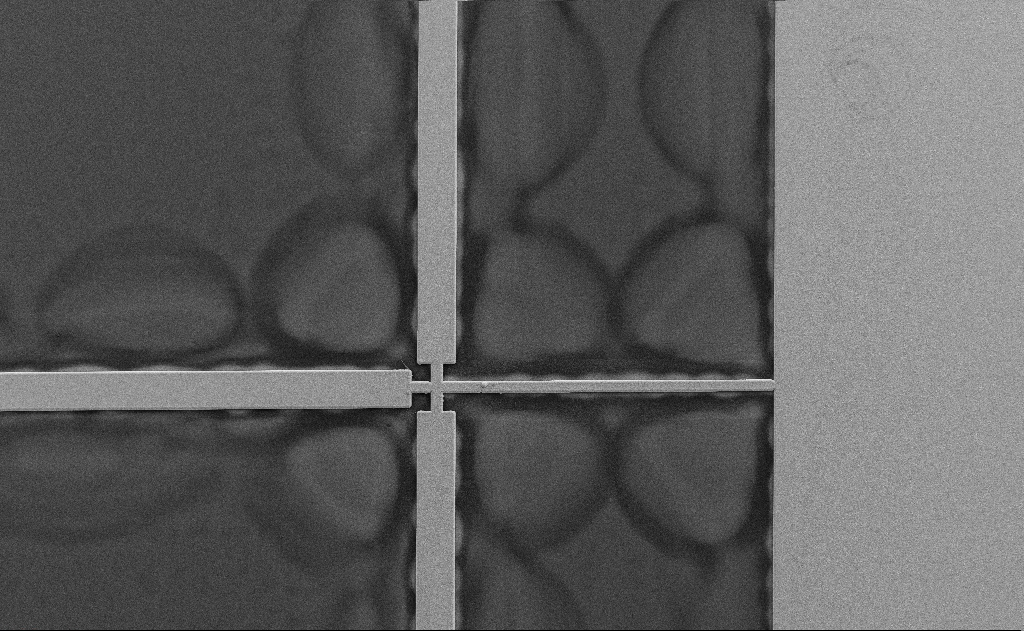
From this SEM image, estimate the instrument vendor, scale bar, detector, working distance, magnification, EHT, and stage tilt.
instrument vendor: Zeiss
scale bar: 100000 nm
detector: SE2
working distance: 6 mm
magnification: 0.247 K X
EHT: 10 kV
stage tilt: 0°